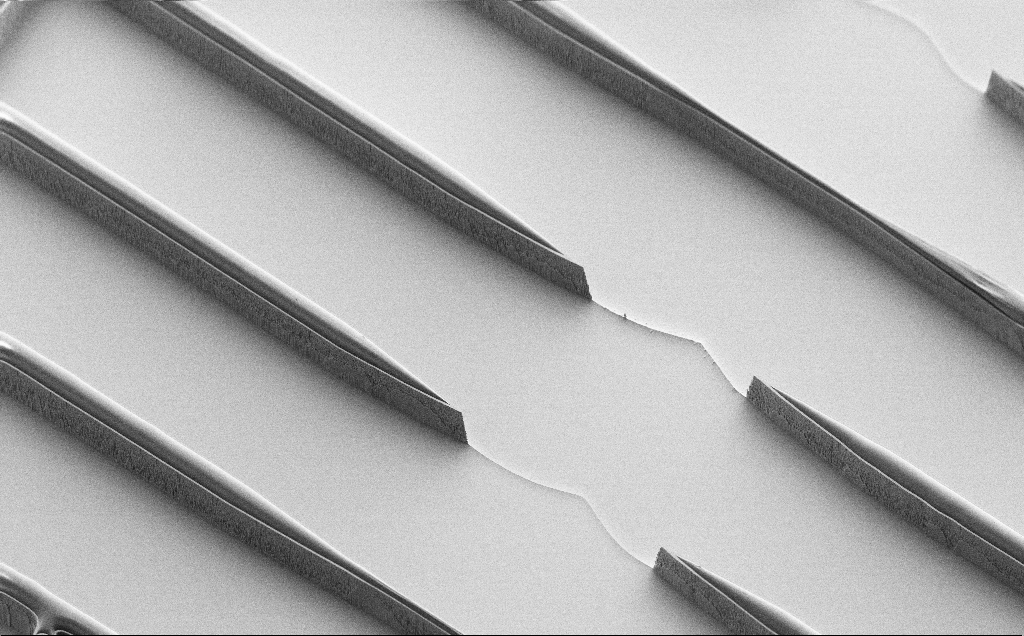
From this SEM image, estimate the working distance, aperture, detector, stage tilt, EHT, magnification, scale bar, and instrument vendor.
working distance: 7 mm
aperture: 30 µm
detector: SE2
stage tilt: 45°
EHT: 1.2 kV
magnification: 0.666 K X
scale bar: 100000 nm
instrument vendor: Zeiss